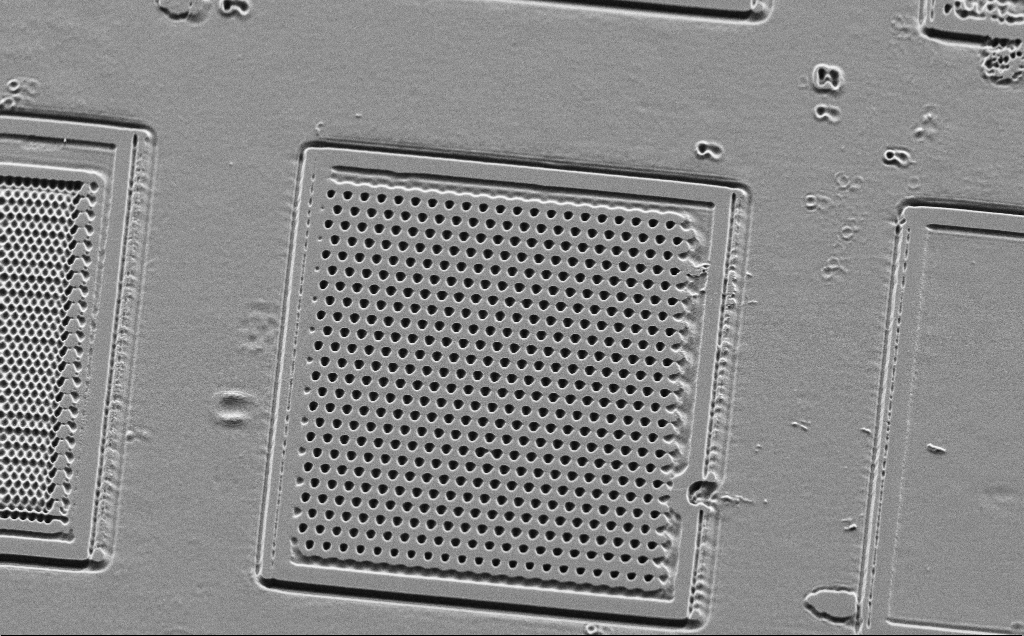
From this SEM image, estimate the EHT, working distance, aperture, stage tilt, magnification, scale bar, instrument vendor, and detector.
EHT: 5 kV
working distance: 10 mm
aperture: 30 µm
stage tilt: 45°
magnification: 1.56 K X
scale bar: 10000 nm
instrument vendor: Zeiss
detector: SE2